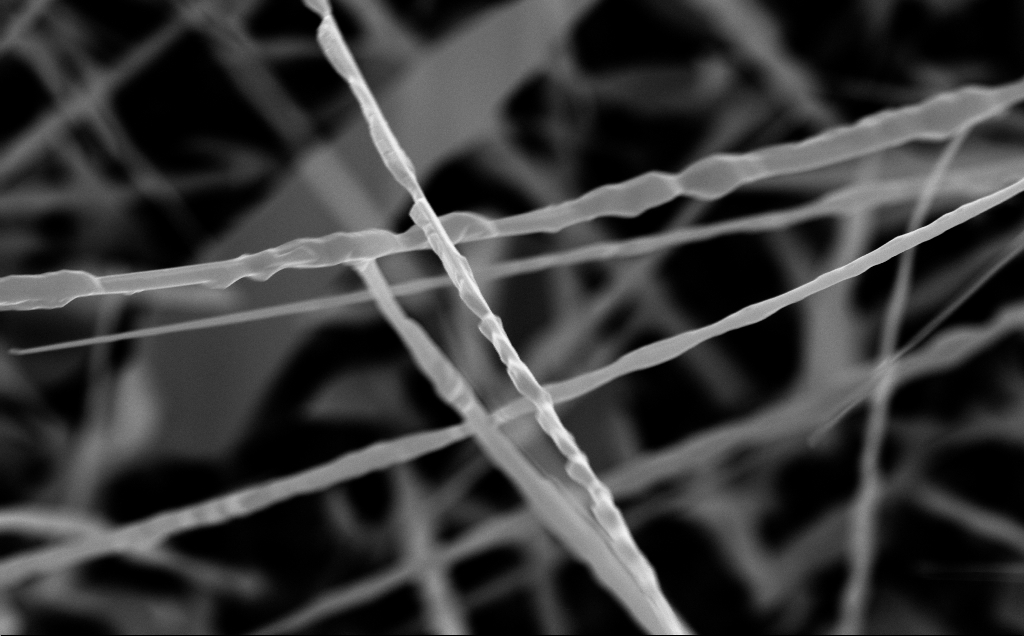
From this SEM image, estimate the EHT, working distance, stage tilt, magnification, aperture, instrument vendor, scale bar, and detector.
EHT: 10 kV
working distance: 6 mm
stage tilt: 0°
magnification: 56.37 K X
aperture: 30 µm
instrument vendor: Zeiss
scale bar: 1000 nm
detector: InLens